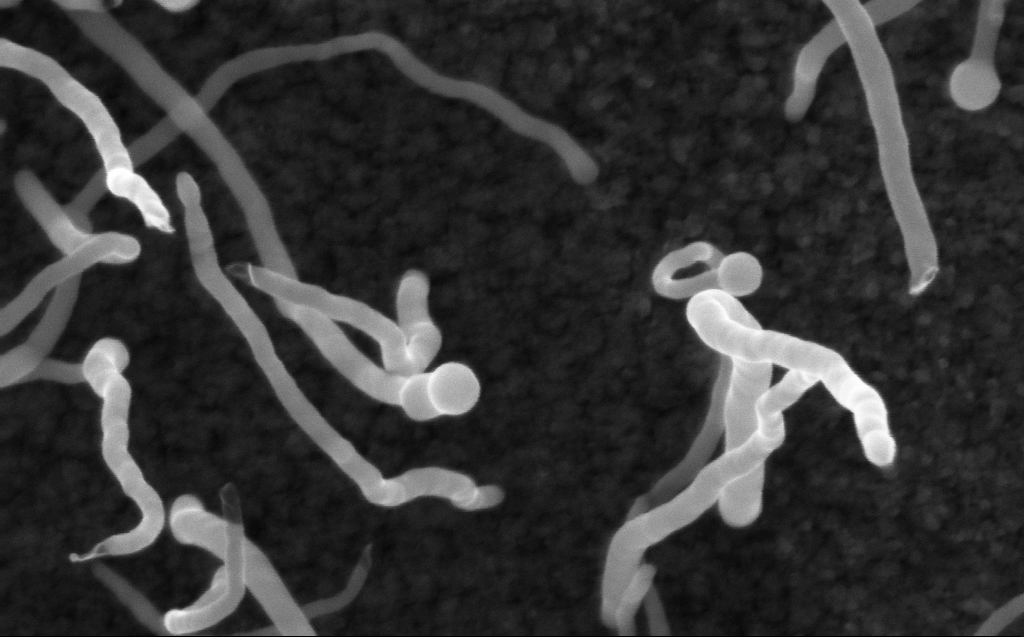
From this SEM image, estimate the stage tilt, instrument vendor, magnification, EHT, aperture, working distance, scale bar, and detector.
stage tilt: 0°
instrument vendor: Zeiss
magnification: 200 K X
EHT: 10 kV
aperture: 30 µm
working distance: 3 mm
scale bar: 200 nm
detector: InLens